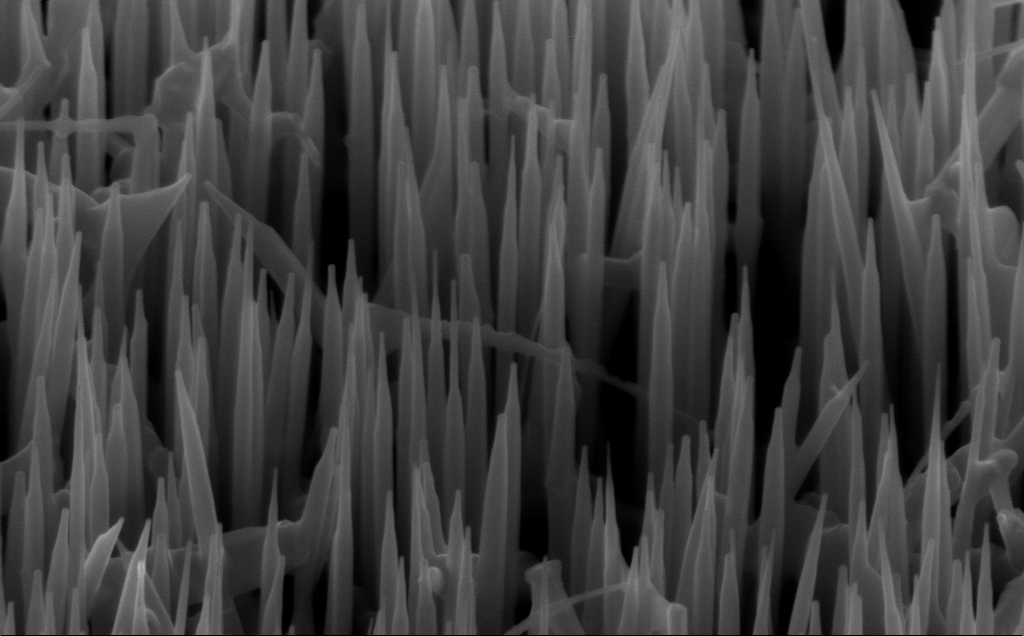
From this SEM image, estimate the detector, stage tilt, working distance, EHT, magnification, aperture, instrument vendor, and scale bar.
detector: InLens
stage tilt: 45°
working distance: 6 mm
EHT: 10 kV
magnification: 80 K X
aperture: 30 µm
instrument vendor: Zeiss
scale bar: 200 nm